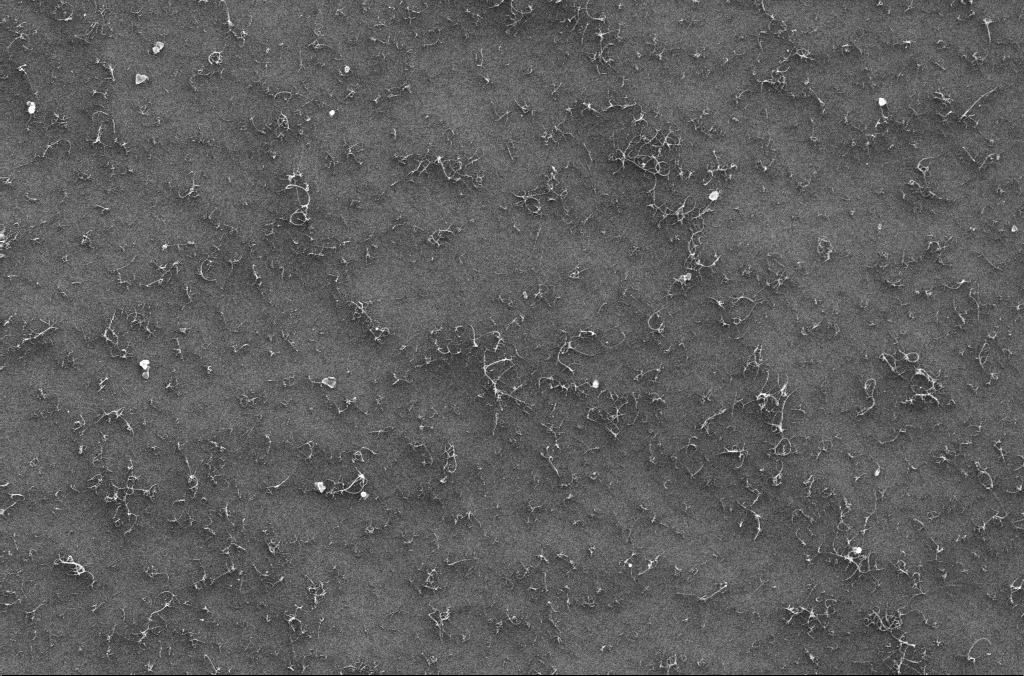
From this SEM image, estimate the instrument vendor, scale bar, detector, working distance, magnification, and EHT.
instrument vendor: Zeiss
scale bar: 1000 nm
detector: InLens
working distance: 3.4 mm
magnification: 32.29 K X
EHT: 10 kV